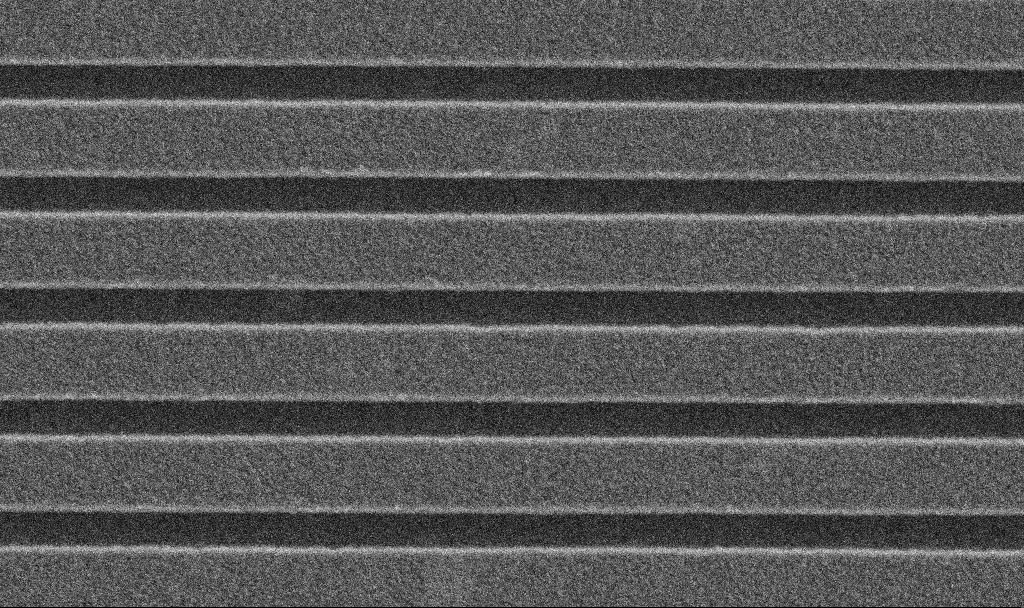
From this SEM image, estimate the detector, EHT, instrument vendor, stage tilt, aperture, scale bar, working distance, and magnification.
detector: SE2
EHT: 5 kV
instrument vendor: Zeiss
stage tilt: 0°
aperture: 30 µm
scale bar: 1000 nm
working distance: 2.9 mm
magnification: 58.86 K X